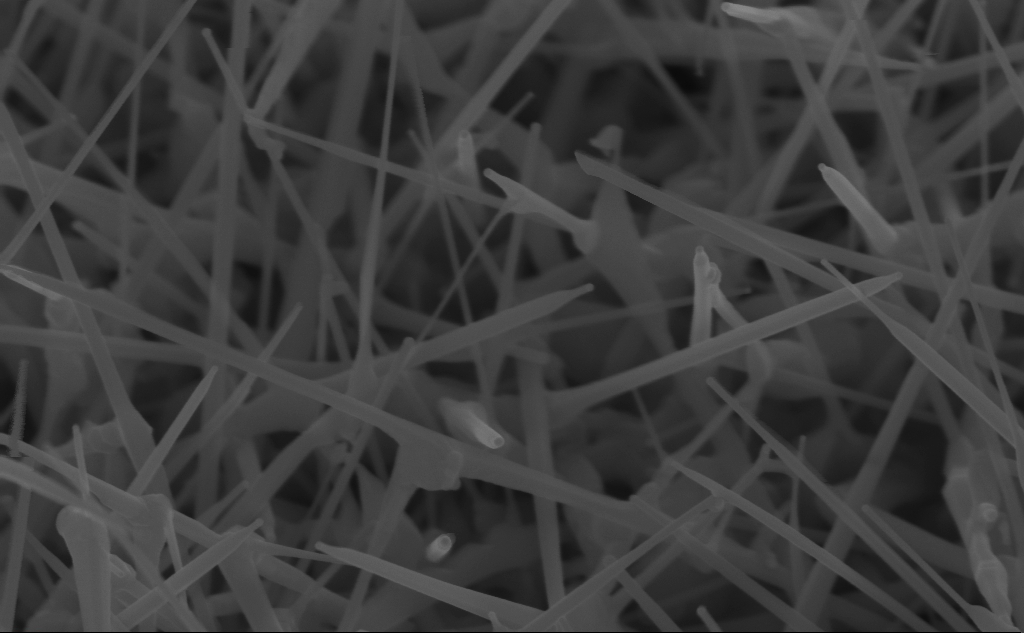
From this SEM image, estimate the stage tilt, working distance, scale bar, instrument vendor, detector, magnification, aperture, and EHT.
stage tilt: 45°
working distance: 5 mm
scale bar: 200 nm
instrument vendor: Zeiss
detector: InLens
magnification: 102.43 K X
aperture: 30 µm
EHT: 10 kV